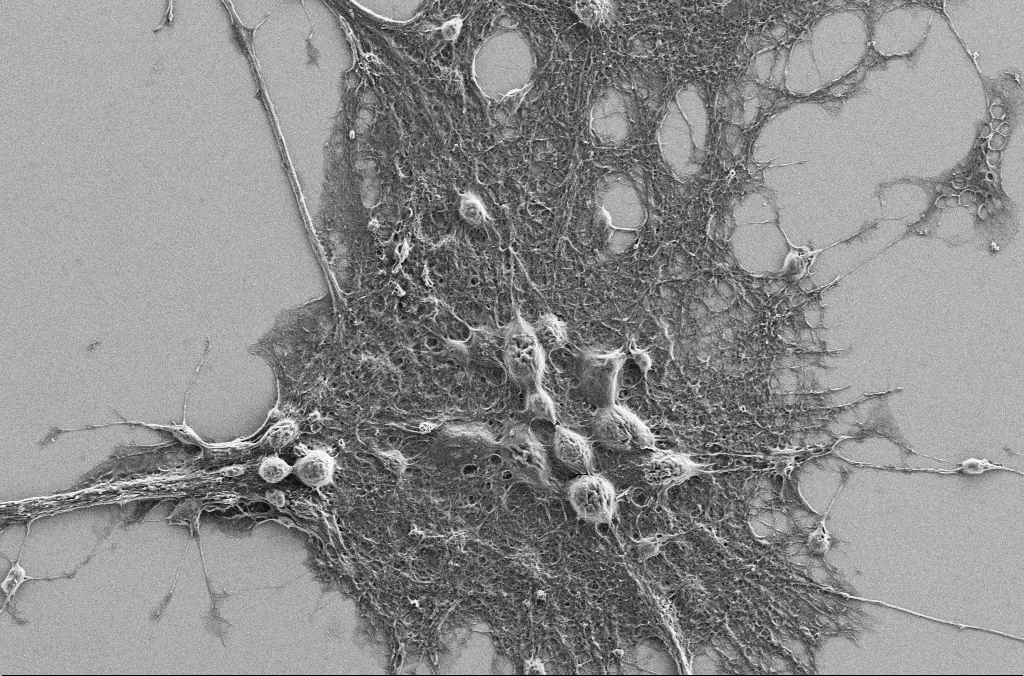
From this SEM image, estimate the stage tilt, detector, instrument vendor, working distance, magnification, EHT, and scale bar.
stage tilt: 0°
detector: SE2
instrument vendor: Zeiss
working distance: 3.2 mm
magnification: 1.5 K X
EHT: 5 kV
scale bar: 10000 nm